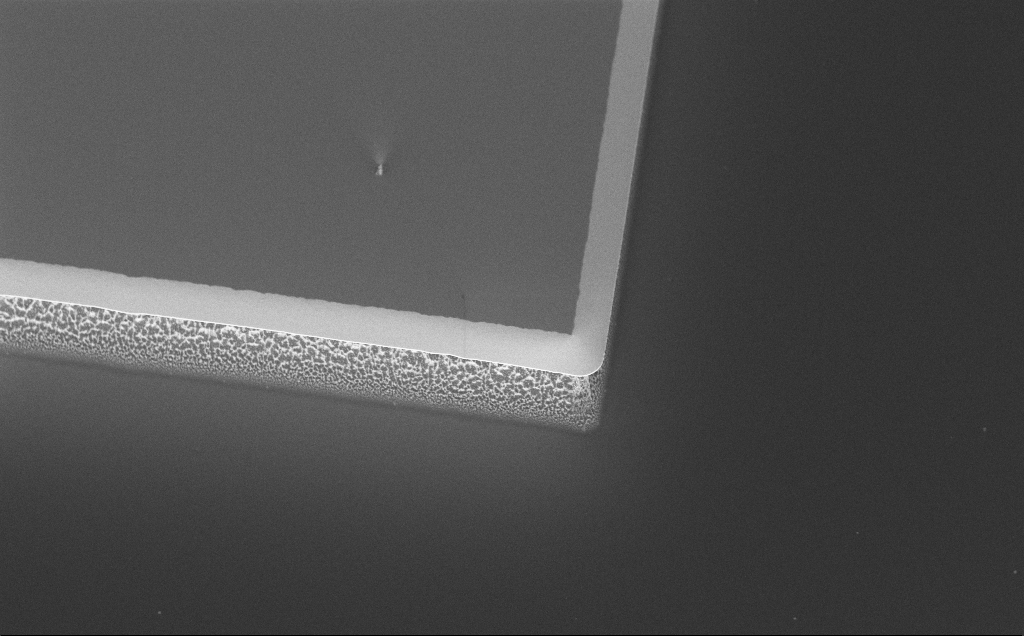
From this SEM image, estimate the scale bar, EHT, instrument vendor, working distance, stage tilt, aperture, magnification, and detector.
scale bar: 20000 nm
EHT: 5 kV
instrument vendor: Zeiss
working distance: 8 mm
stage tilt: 45°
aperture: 30 µm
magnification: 1.9 K X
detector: InLens